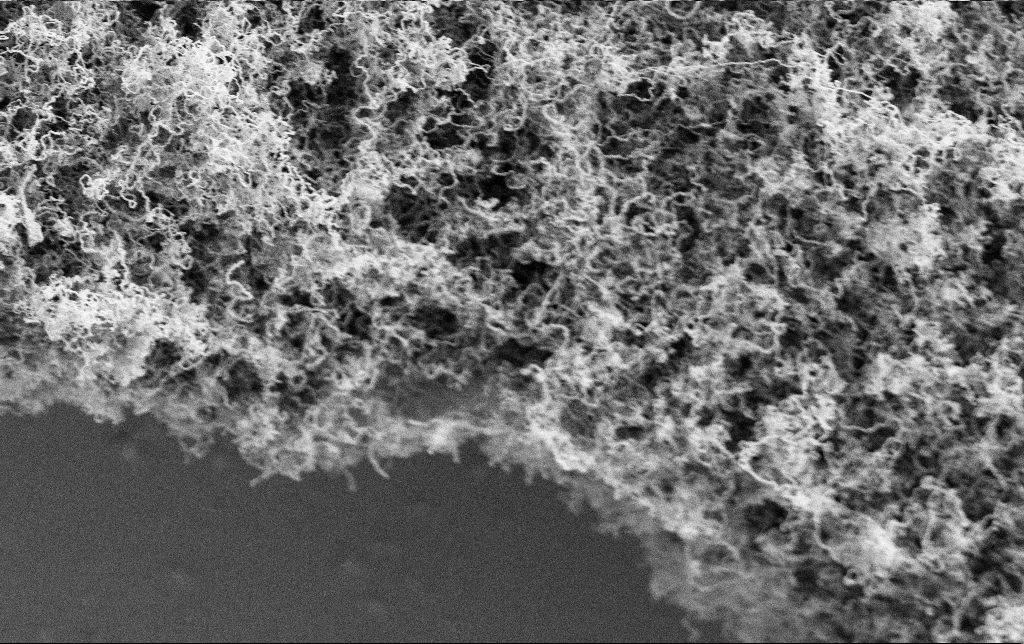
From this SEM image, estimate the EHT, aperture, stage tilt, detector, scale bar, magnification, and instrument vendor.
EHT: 2 kV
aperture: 30 µm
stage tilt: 0°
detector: SE2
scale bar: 2000 nm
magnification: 10 K X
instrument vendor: Zeiss